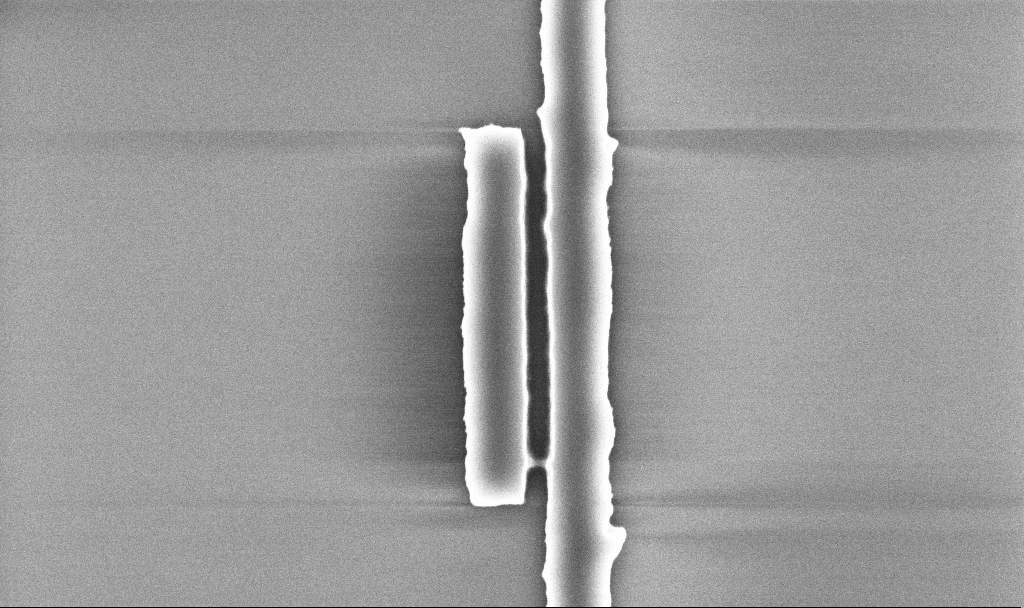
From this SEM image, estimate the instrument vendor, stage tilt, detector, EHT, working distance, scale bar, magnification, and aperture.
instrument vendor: Zeiss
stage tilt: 0°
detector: InLens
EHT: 5 kV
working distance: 5.2 mm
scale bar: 1000 nm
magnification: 46.98 K X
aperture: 30 µm